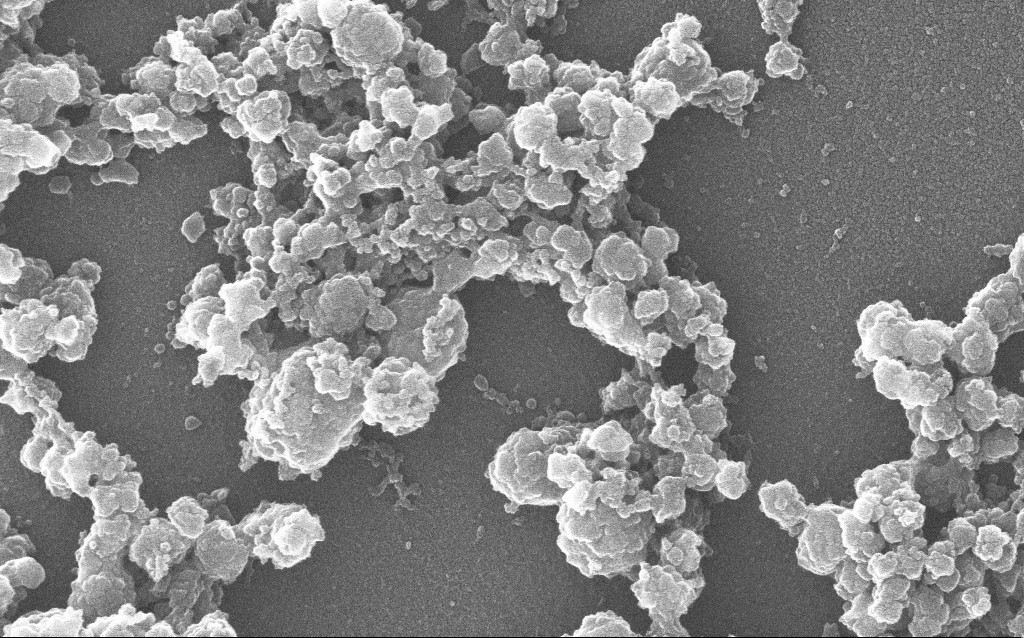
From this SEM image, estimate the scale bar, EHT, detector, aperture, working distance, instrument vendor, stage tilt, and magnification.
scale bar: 200 nm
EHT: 20 kV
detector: InLens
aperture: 30 µm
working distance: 1.7 mm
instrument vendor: Zeiss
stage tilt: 0°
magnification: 100 K X